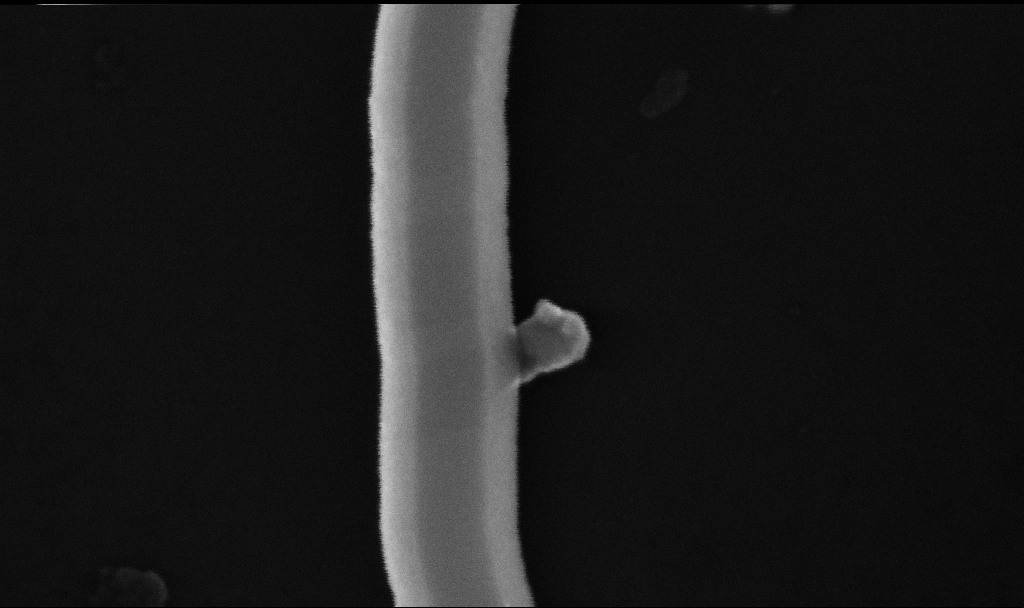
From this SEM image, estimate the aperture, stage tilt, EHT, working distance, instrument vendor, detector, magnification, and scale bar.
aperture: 30 µm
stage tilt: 0°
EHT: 10 kV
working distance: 6.7 mm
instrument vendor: Zeiss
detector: InLens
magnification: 456.3 K X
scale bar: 100 nm